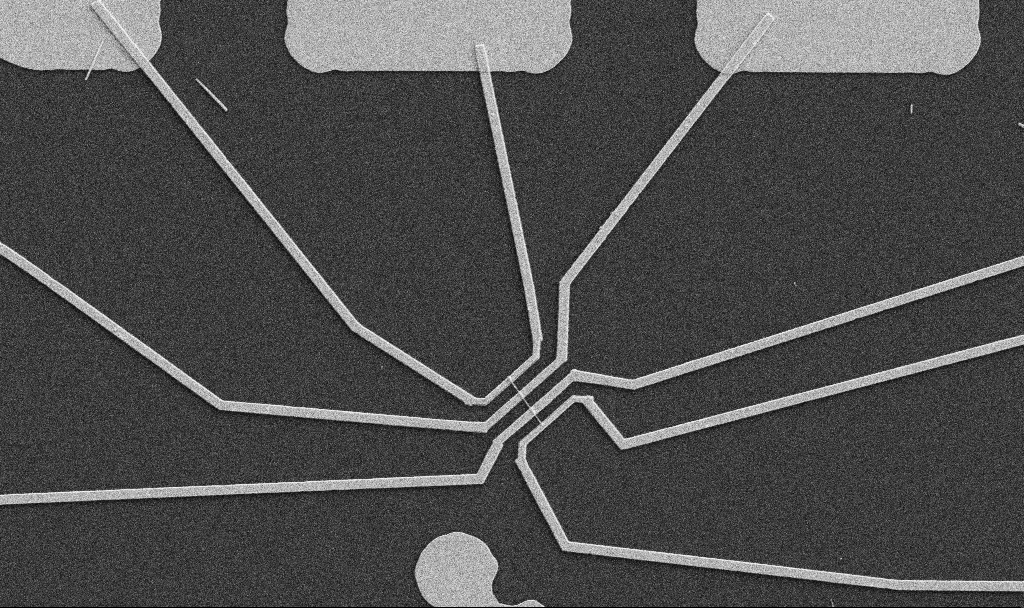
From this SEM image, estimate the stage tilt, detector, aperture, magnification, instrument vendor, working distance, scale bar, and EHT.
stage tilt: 0°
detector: SE2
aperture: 30 µm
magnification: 5 K X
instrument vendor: Zeiss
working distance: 10.7 mm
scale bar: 10000 nm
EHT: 5 kV